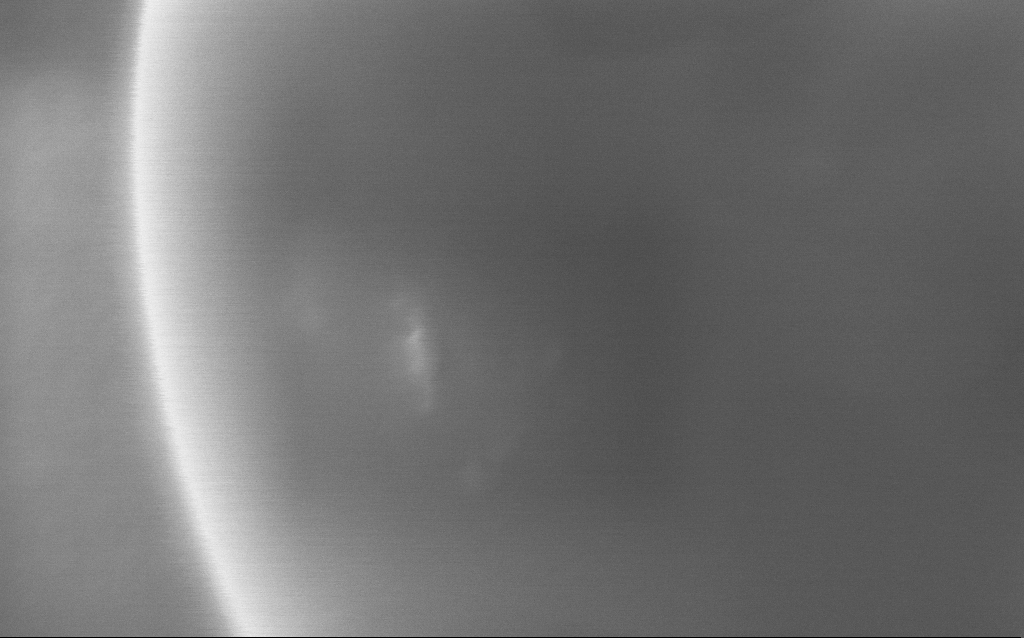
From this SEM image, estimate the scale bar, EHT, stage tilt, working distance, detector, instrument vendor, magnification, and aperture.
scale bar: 100 nm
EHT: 5 kV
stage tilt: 0°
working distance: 3 mm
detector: InLens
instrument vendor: Zeiss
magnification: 531.5 K X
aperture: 30 µm